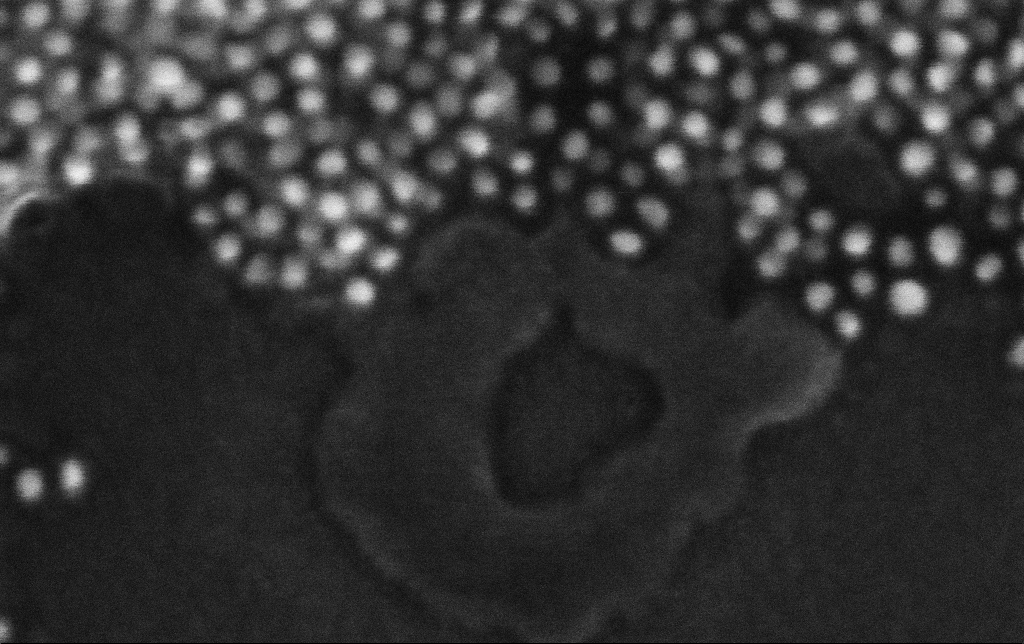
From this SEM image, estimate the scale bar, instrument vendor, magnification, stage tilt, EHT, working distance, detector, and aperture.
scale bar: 20 nm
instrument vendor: Zeiss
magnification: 906.31 K X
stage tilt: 0°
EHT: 10 kV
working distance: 3.4 mm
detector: InLens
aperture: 30 µm